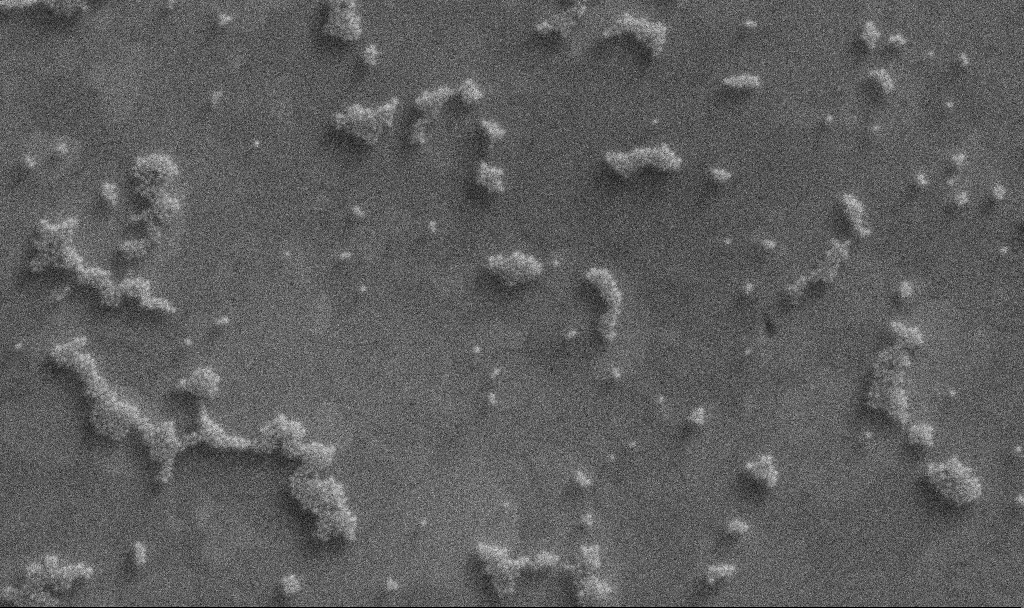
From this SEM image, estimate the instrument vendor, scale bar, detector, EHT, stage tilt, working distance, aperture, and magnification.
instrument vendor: Zeiss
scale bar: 200 nm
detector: InLens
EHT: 5 kV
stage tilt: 0°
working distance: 3.3 mm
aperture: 30 µm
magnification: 131.49 K X